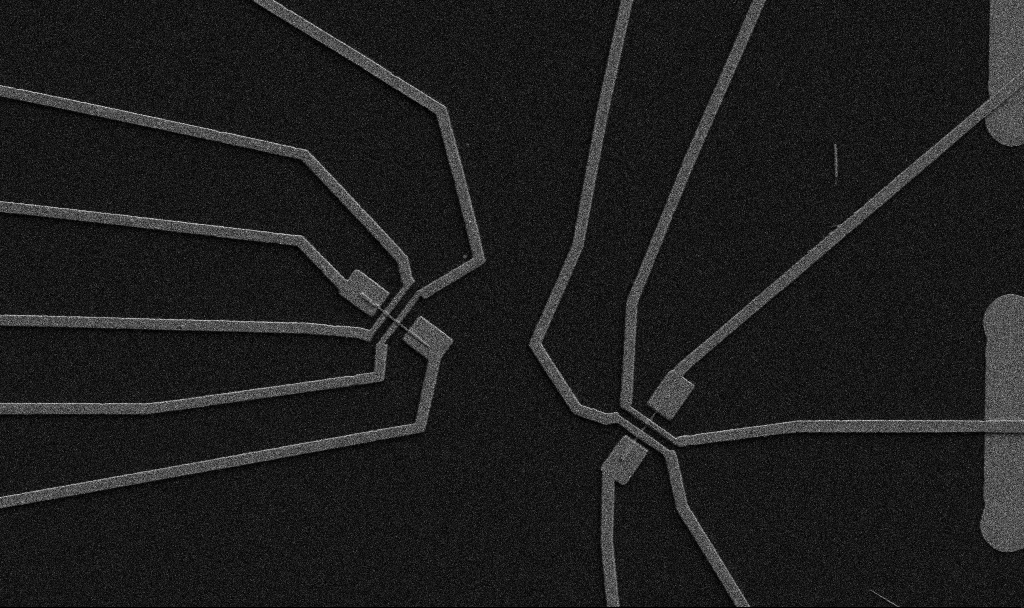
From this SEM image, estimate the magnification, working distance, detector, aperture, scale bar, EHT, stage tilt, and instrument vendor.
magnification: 5 K X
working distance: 10.7 mm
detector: SE2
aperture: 30 µm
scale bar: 10000 nm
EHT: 5 kV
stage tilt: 0°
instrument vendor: Zeiss